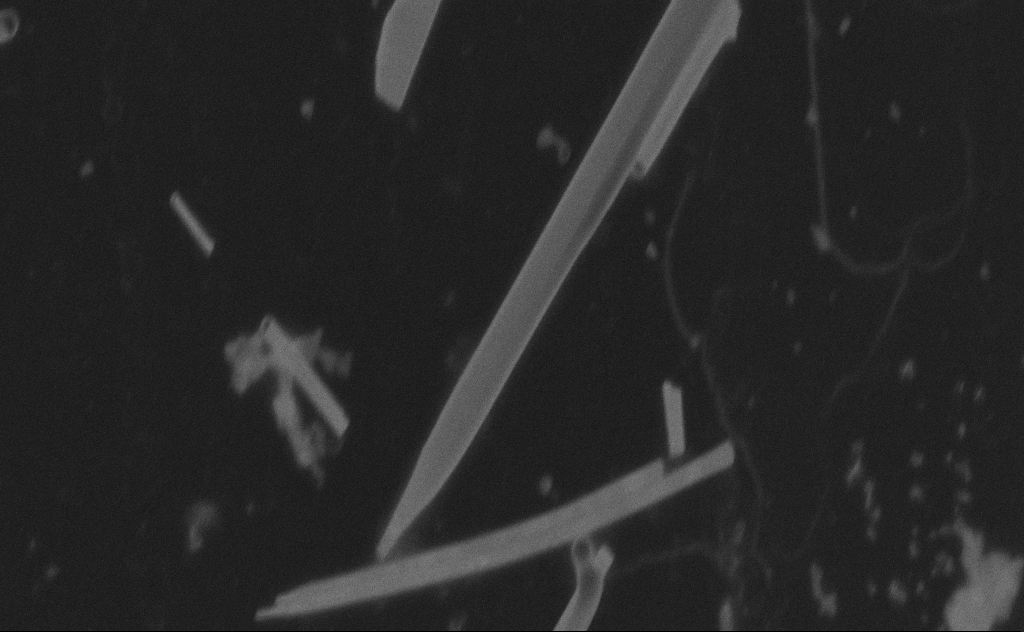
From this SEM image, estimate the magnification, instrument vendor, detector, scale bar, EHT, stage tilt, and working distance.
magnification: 103.29 K X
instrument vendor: Zeiss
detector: SE2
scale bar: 200 nm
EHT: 20 kV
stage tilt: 0°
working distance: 9 mm